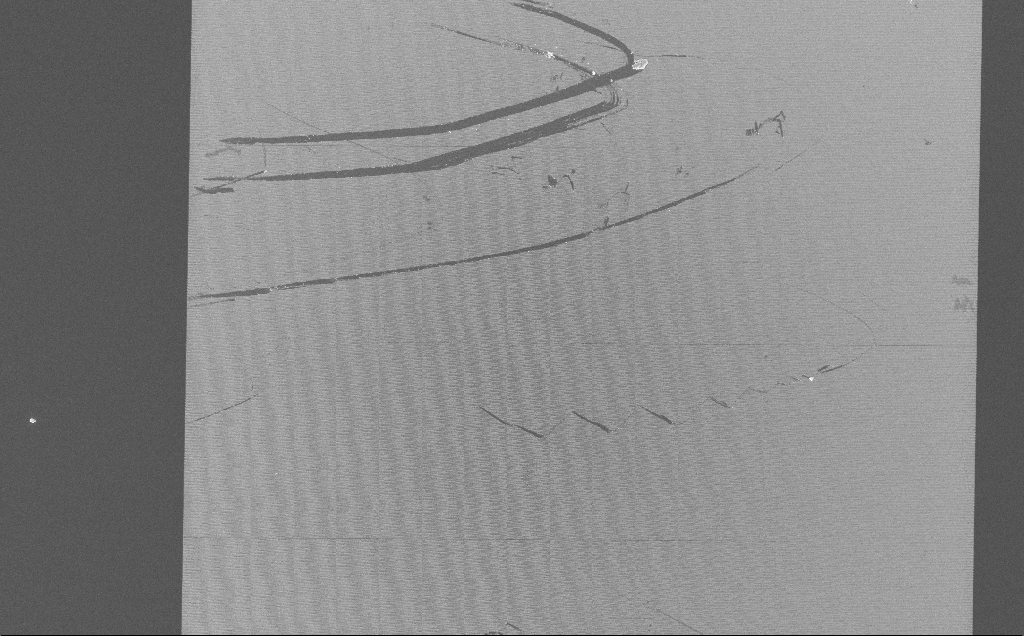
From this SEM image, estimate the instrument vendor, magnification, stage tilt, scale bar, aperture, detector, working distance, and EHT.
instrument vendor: Zeiss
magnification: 0.294 K X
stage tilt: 0°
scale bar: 100000 nm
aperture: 30 µm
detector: InLens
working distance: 7 mm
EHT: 10 kV